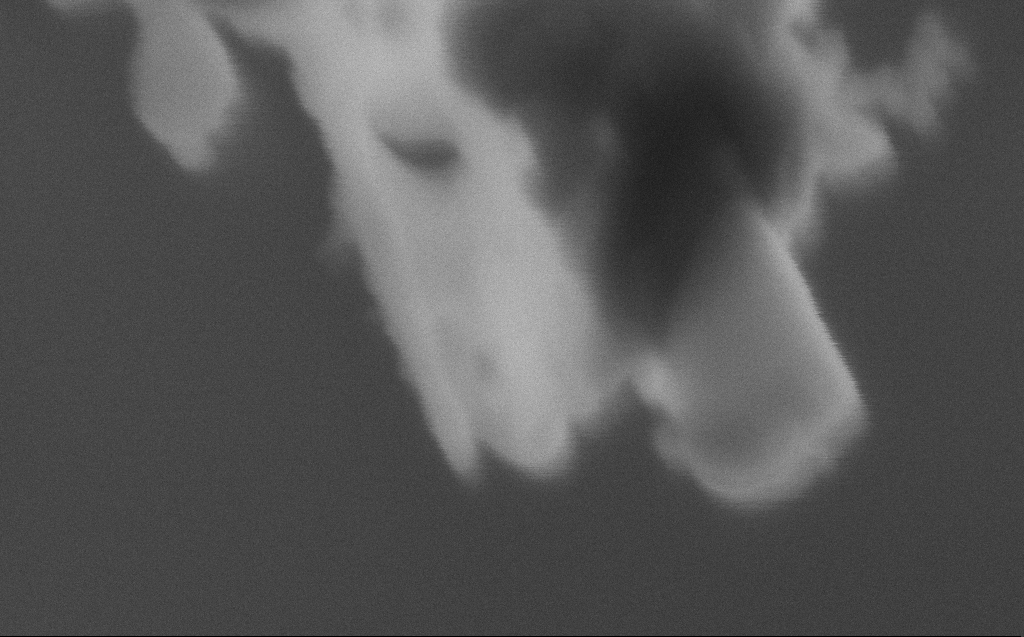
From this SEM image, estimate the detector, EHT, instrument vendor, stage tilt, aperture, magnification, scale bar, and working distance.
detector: SE2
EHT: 5 kV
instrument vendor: Zeiss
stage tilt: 45°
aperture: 30 µm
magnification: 580.47 K X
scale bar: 100 nm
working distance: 4 mm